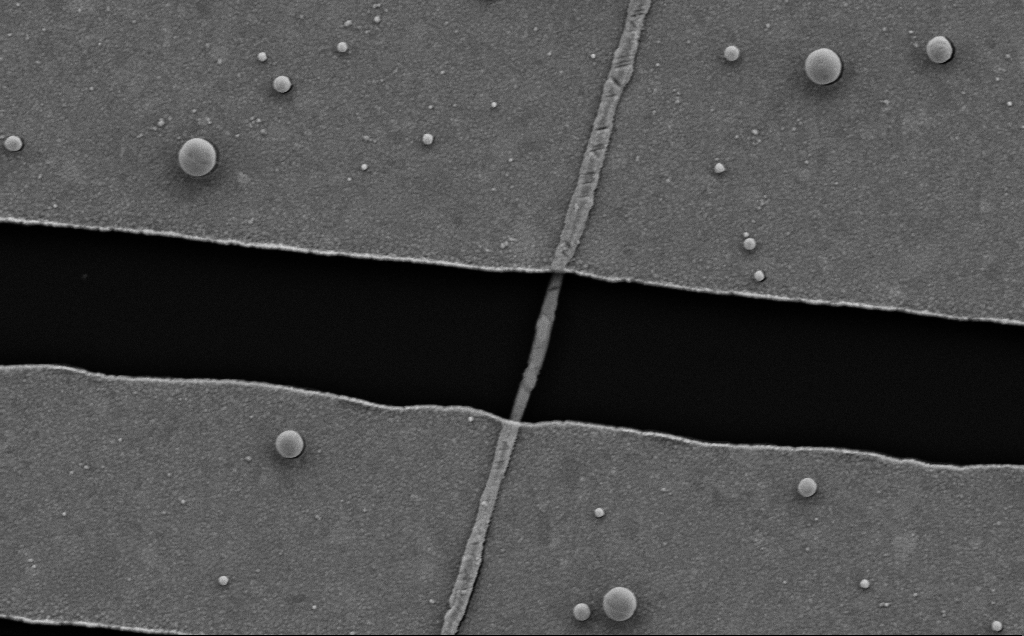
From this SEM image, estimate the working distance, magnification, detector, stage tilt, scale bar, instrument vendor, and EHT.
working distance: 8 mm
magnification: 35.11 K X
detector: SE2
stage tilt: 0°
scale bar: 1000 nm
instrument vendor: Zeiss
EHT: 5 kV